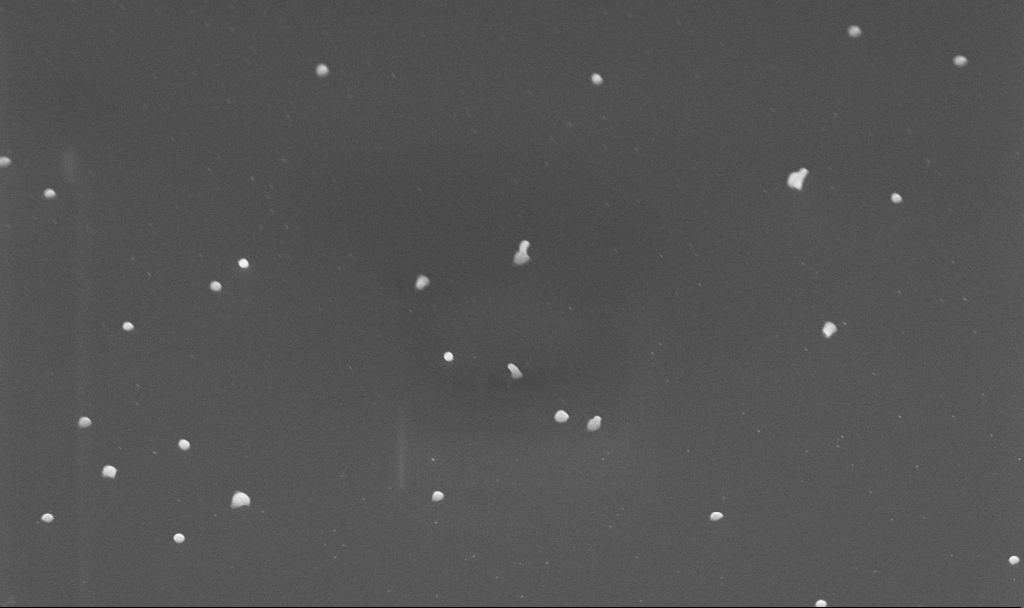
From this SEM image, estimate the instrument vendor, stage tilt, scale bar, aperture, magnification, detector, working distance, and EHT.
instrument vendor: Zeiss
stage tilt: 45°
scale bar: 1000 nm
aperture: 30 µm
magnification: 50 K X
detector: InLens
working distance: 4.7 mm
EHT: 10 kV